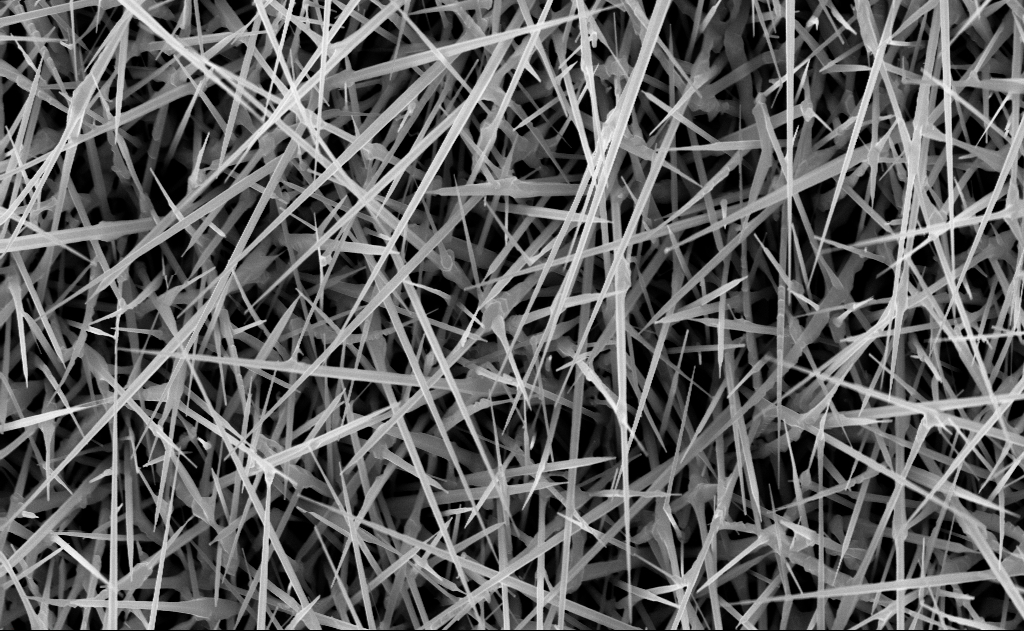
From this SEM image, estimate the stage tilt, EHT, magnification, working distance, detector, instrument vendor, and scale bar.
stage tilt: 0°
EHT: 10 kV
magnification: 20 K X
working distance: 10 mm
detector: InLens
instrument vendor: Zeiss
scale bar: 2000 nm